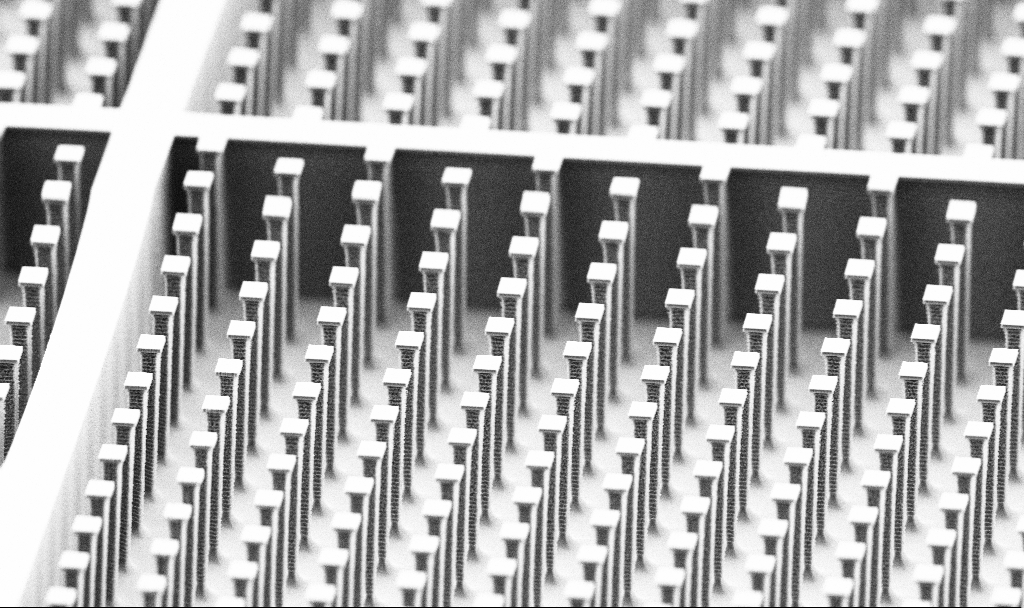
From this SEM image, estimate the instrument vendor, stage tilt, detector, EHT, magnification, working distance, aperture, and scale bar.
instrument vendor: Zeiss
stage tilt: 70°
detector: SE2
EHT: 5 kV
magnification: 3.11 K X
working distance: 4.5 mm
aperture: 30 µm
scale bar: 10000 nm